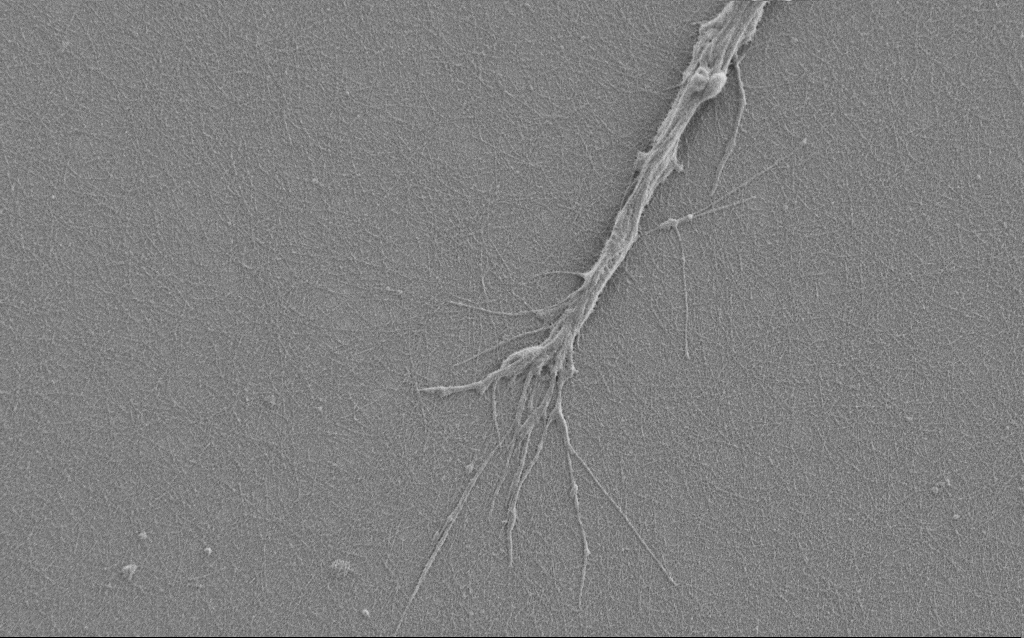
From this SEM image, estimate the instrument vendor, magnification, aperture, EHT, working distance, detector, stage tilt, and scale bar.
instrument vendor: Zeiss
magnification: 7.5 K X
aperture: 30 µm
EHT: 1 kV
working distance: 6 mm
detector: SE2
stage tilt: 0°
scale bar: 2000 nm